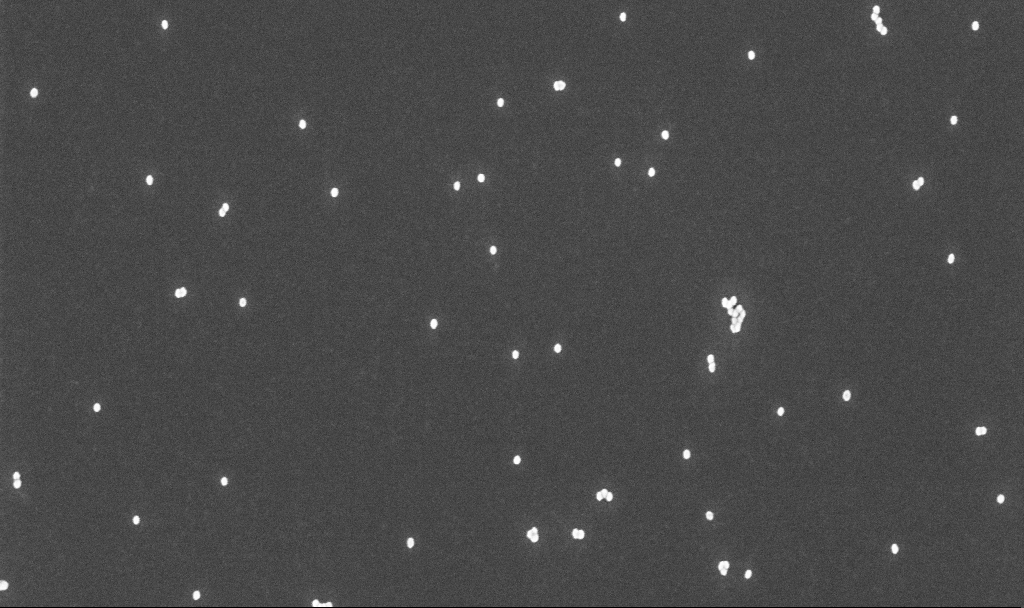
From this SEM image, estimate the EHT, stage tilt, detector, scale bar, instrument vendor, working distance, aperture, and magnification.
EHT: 10 kV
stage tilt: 0°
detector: InLens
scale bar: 200 nm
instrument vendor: Zeiss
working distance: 4.8 mm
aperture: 30 µm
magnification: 100 K X